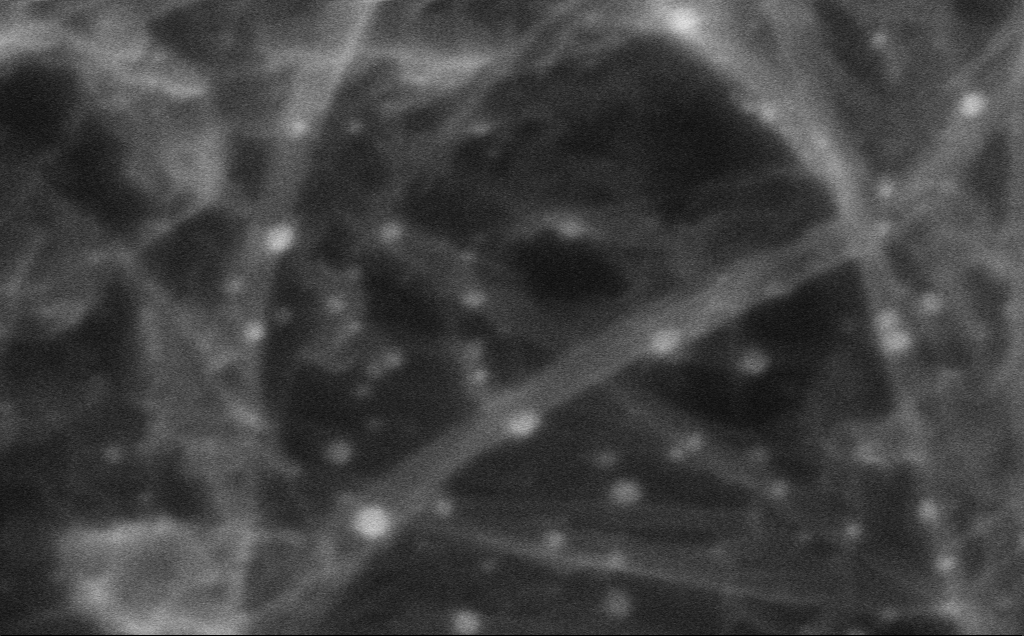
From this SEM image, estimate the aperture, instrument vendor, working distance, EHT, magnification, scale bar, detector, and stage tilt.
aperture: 30 µm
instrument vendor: Zeiss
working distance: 3 mm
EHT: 10 kV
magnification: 978.84 K X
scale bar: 20 nm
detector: InLens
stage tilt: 0°